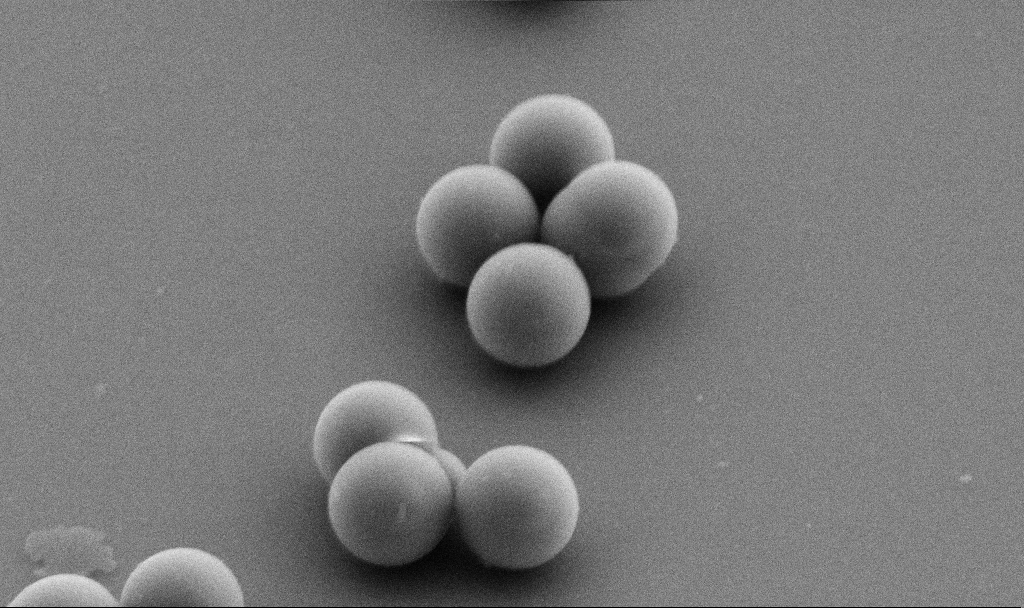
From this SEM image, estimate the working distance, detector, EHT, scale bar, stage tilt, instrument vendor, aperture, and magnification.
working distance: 7.7 mm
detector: SE2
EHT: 5 kV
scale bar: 2000 nm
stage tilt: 45°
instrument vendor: Zeiss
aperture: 30 µm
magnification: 35.73 K X